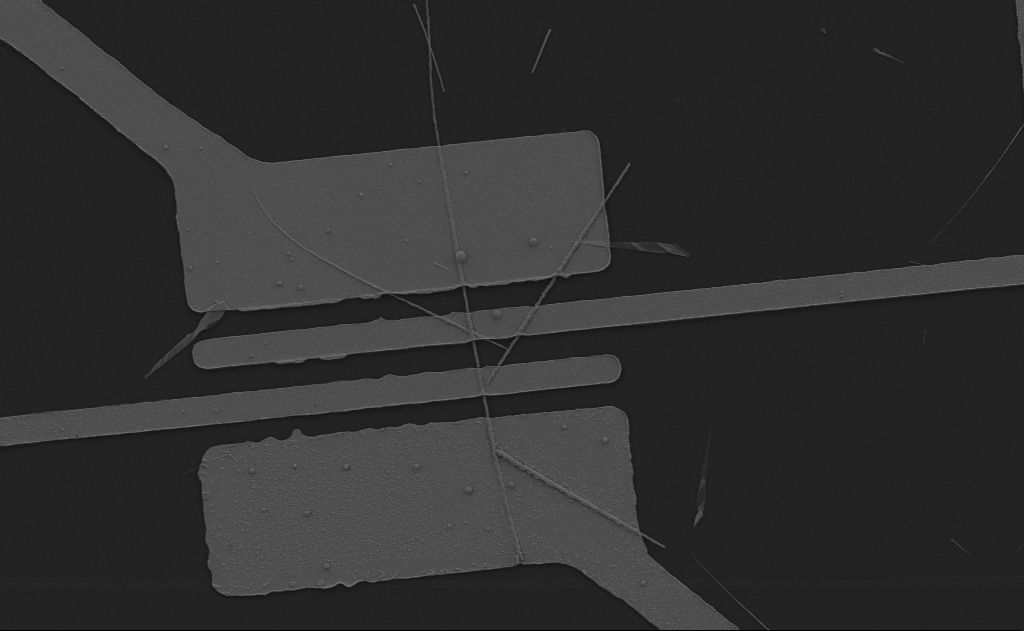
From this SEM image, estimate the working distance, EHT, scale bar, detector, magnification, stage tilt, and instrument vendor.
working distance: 12 mm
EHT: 5 kV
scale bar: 10000 nm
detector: SE2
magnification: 5.2 K X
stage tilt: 0°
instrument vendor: Zeiss